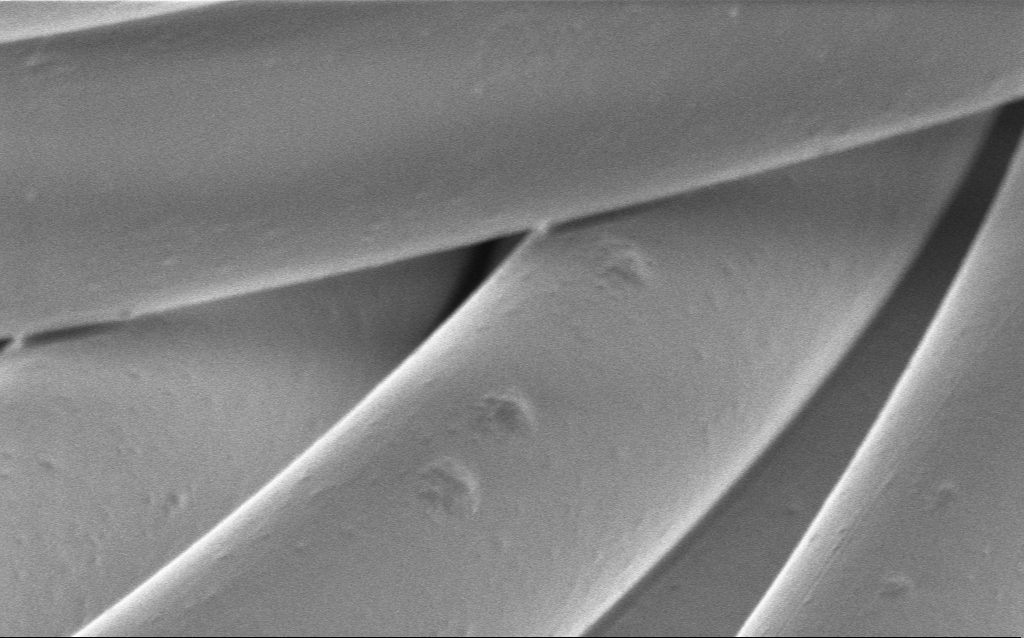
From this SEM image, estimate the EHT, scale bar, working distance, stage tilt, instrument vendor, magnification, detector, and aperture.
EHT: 1 kV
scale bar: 10000 nm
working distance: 4 mm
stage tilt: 0°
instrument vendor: Zeiss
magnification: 5.15 K X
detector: InLens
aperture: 30 µm